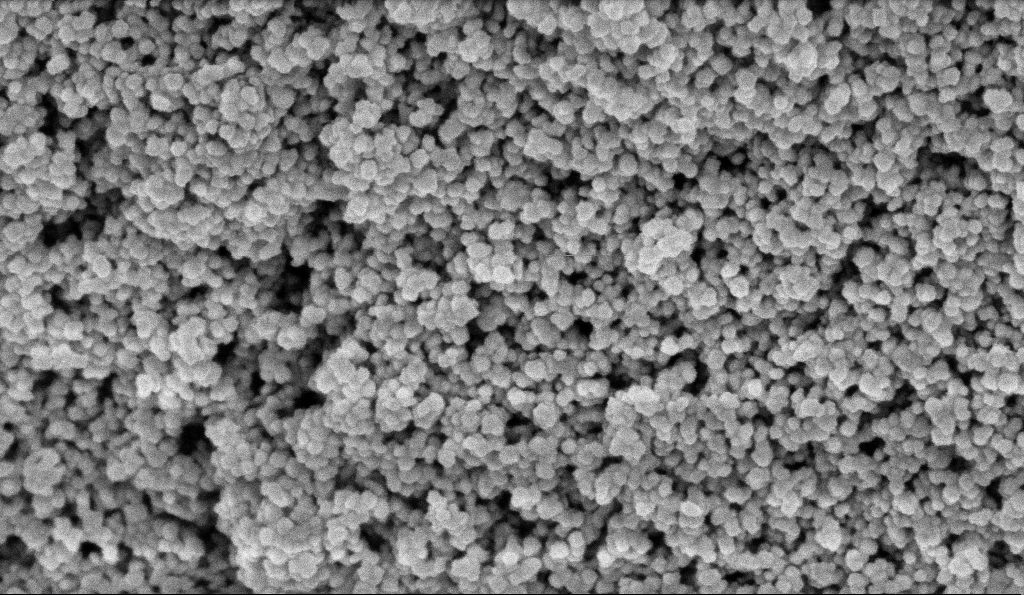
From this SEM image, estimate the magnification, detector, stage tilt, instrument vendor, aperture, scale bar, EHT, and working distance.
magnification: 135 K X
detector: InLens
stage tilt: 0°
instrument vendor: Zeiss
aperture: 30 µm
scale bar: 100 nm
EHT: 5 kV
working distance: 6 mm